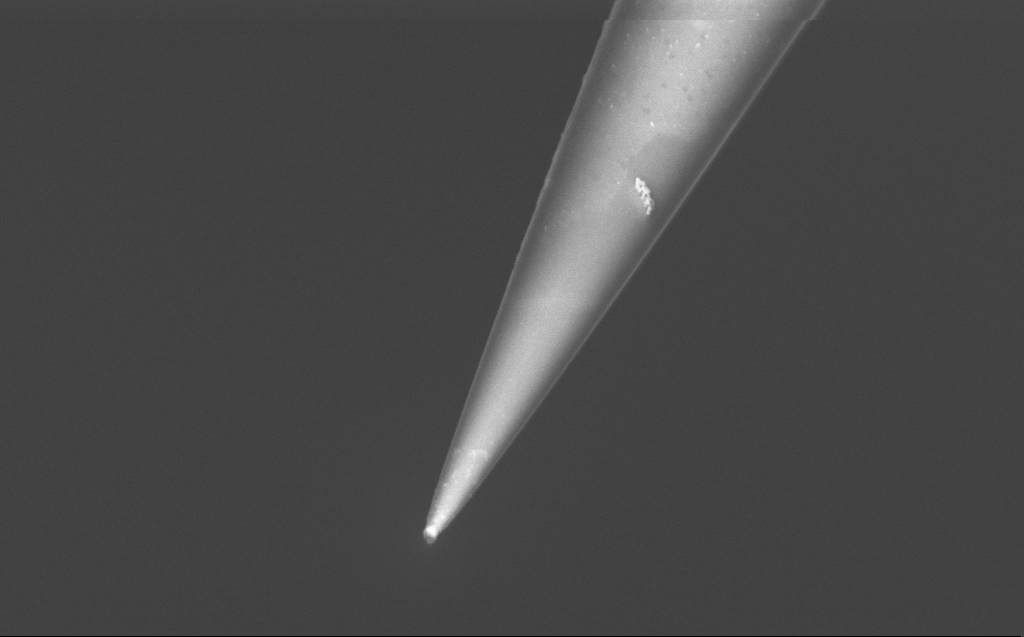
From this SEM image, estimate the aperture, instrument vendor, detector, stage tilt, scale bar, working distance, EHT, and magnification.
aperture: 30 µm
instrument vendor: Zeiss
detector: InLens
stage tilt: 45°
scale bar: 1000 nm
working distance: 6 mm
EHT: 2 kV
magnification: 50 K X